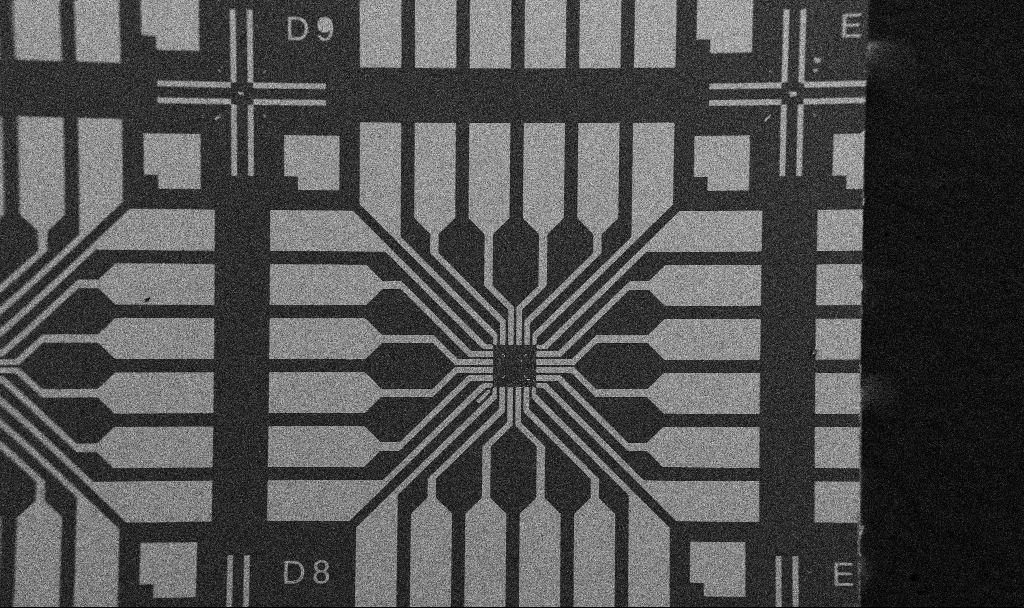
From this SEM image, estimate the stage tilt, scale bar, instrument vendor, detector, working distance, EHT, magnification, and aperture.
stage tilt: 0°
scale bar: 200000 nm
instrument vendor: Zeiss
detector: SE2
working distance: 10.7 mm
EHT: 5 kV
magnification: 0.1 K X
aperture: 30 µm